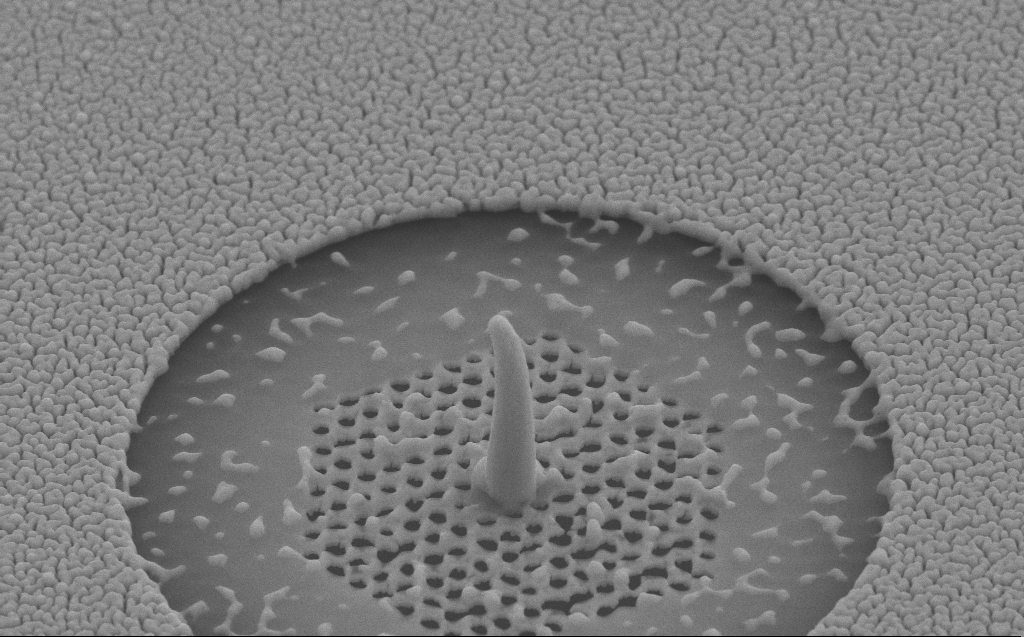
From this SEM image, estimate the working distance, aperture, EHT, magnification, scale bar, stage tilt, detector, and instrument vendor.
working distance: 6 mm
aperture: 30 µm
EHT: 10 kV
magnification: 40.05 K X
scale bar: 1000 nm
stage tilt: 45°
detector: SE2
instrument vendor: Zeiss